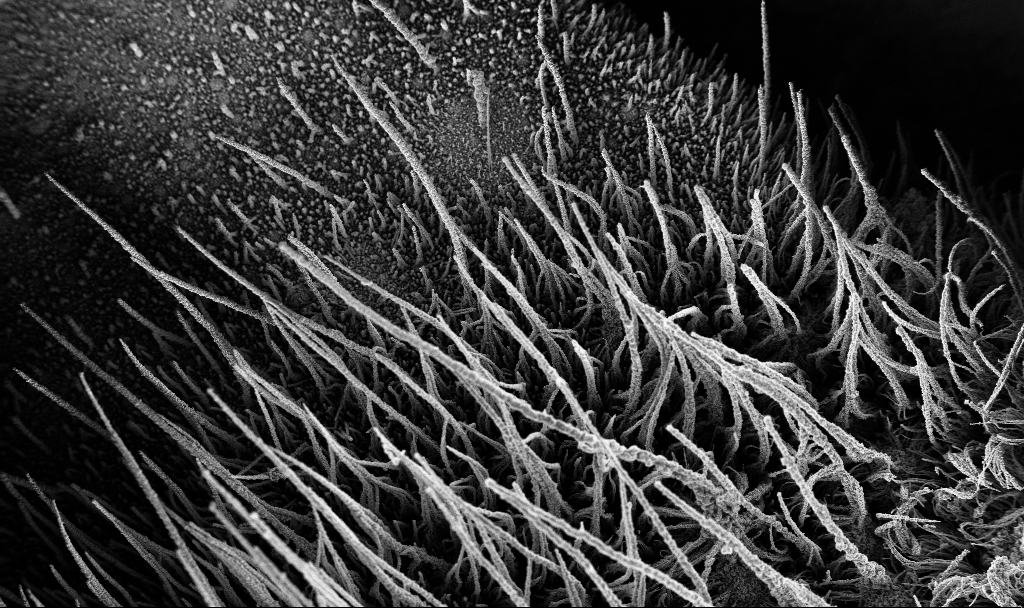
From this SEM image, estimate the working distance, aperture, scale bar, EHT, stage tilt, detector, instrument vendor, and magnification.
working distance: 3.8 mm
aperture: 30 µm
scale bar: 100000 nm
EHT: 3 kV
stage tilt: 0°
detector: InLens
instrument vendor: Zeiss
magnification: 0.15 K X